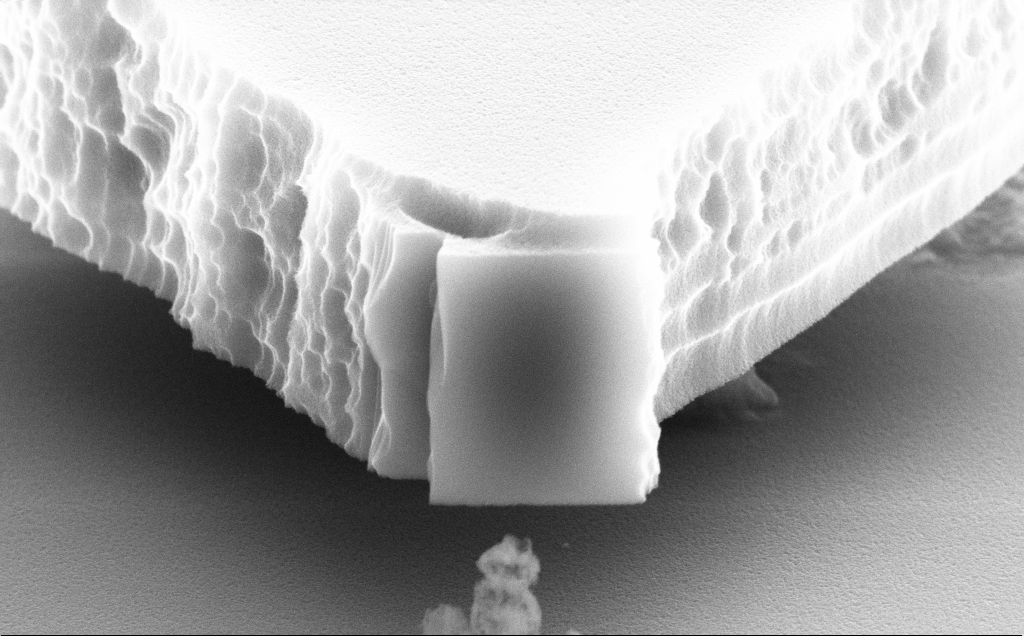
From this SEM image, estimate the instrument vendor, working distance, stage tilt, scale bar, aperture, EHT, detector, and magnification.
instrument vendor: Zeiss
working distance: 9 mm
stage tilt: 70°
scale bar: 1000 nm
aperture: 30 µm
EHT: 10 kV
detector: SE2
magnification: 44.97 K X